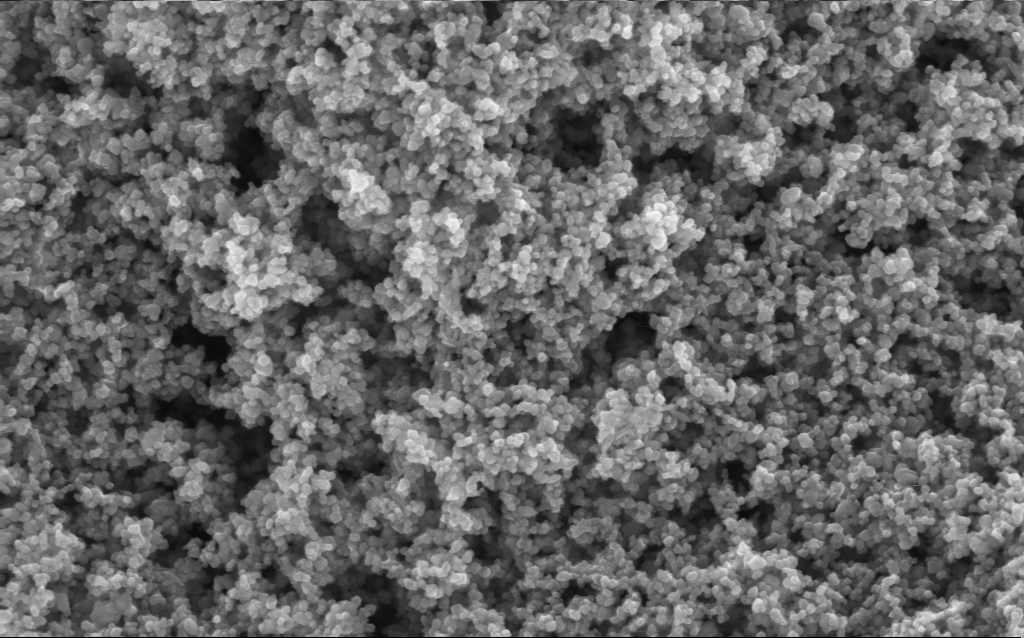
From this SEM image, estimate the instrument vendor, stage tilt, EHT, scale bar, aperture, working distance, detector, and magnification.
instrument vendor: Zeiss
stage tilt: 0°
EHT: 5 kV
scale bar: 100 nm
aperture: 30 µm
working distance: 4.4 mm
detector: InLens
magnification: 130 K X